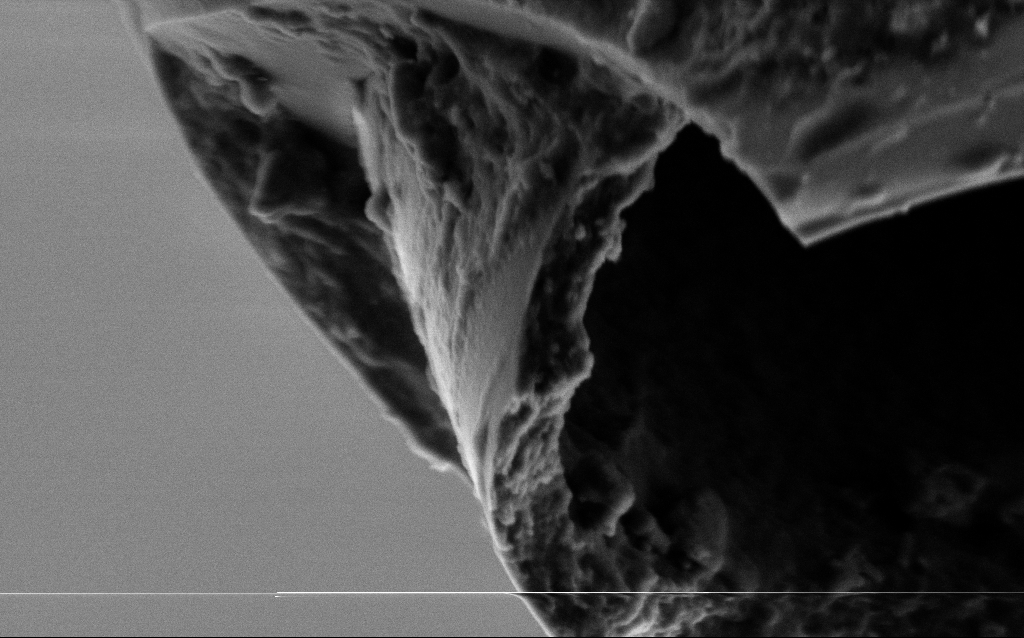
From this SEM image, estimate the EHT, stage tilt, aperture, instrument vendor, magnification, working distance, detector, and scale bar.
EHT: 2 kV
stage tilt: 45°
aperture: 30 µm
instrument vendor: Zeiss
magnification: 75 K X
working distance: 6 mm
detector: SE2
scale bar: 200 nm